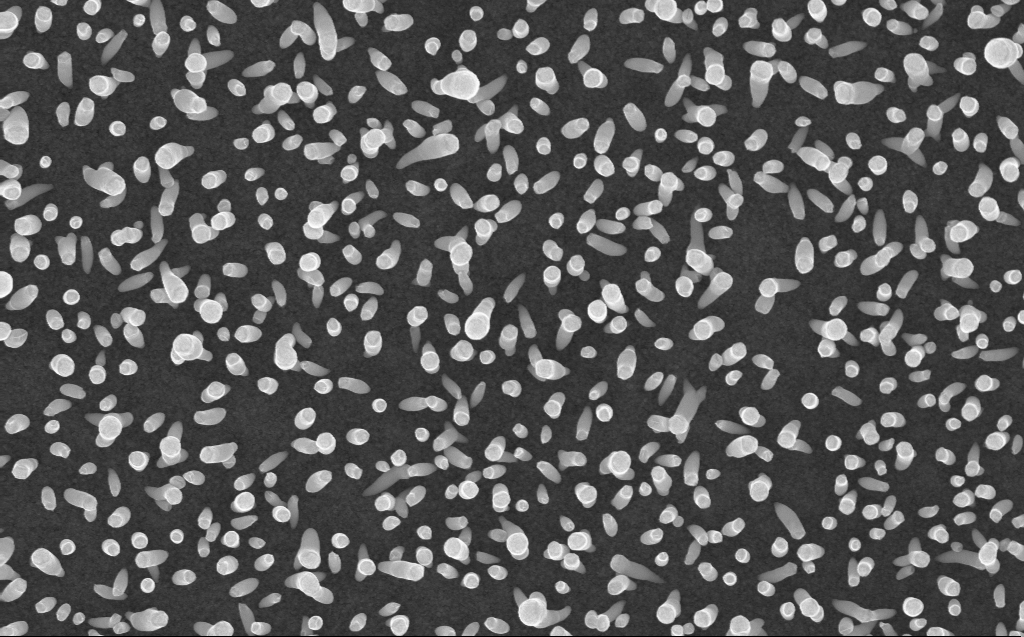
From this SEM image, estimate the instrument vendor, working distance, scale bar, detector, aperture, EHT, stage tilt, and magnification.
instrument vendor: Zeiss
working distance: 3 mm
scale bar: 1000 nm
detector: InLens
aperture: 30 µm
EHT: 10 kV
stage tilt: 0°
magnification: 50 K X